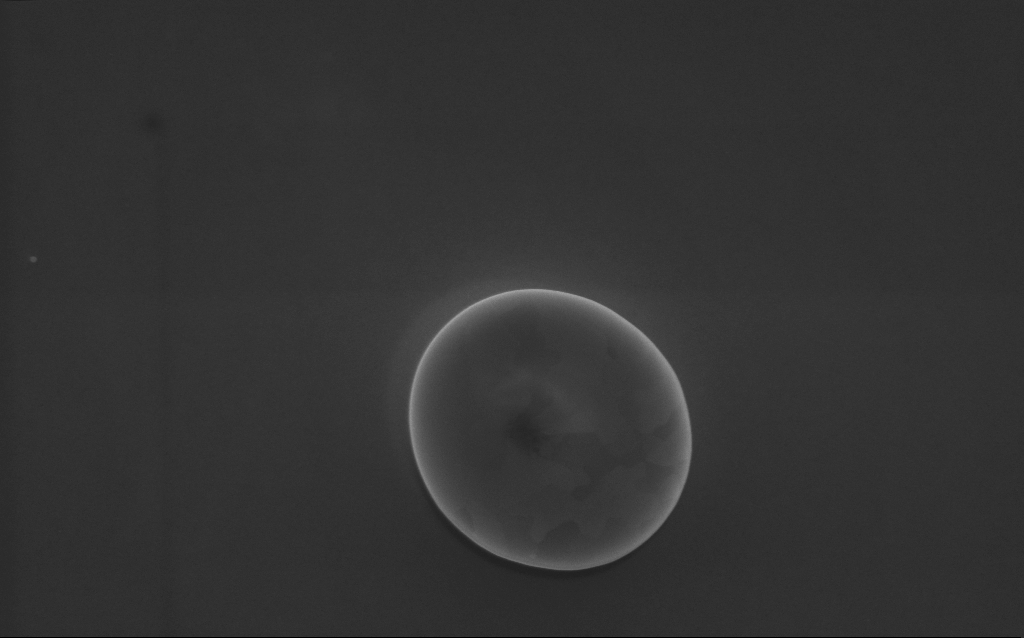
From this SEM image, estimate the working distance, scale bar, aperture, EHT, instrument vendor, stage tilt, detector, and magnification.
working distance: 3 mm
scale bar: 1000 nm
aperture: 30 µm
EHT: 5 kV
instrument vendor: Zeiss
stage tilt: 0°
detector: InLens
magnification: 62 K X